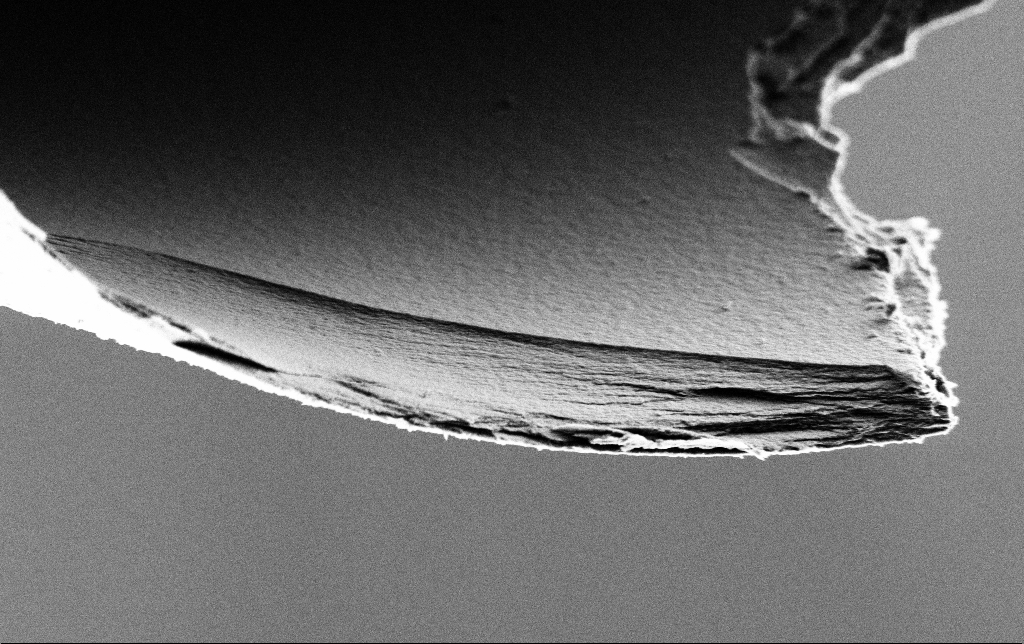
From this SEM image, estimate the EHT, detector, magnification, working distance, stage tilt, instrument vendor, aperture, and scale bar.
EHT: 2 kV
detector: SE2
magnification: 15 K X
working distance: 7.2 mm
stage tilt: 45°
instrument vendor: Zeiss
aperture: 30 µm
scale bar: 2000 nm